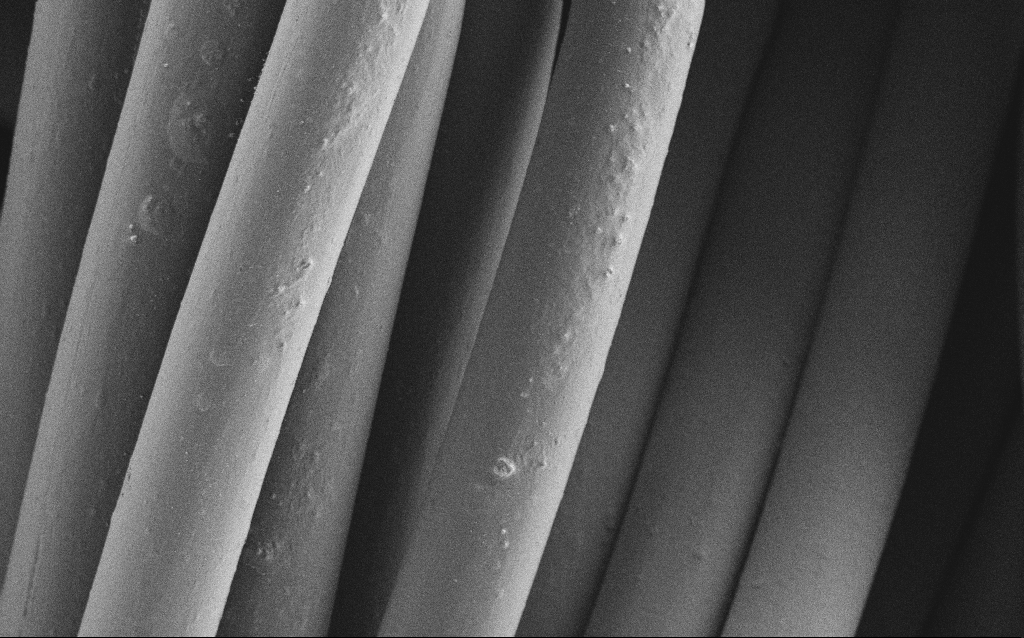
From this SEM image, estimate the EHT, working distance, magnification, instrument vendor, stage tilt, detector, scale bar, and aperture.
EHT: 1 kV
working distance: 4 mm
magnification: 2.07 K X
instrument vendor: Zeiss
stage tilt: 0°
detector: SE2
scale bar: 10000 nm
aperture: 30 µm